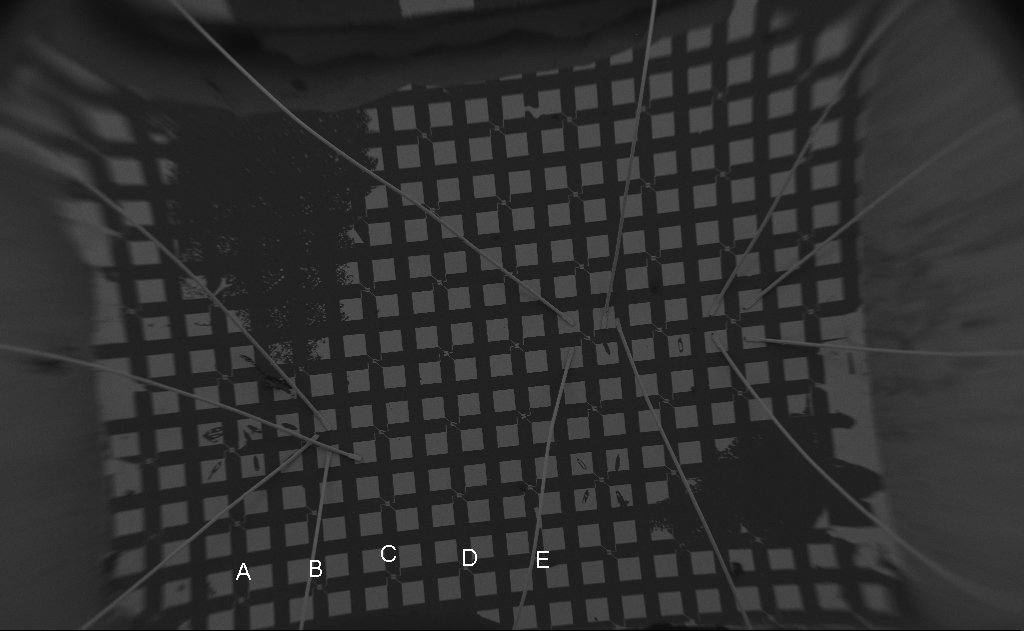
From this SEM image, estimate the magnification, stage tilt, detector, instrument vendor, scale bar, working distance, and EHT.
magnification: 0.054 K X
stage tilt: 0°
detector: SE2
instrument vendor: Zeiss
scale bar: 1e+06 nm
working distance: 13 mm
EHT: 5 kV